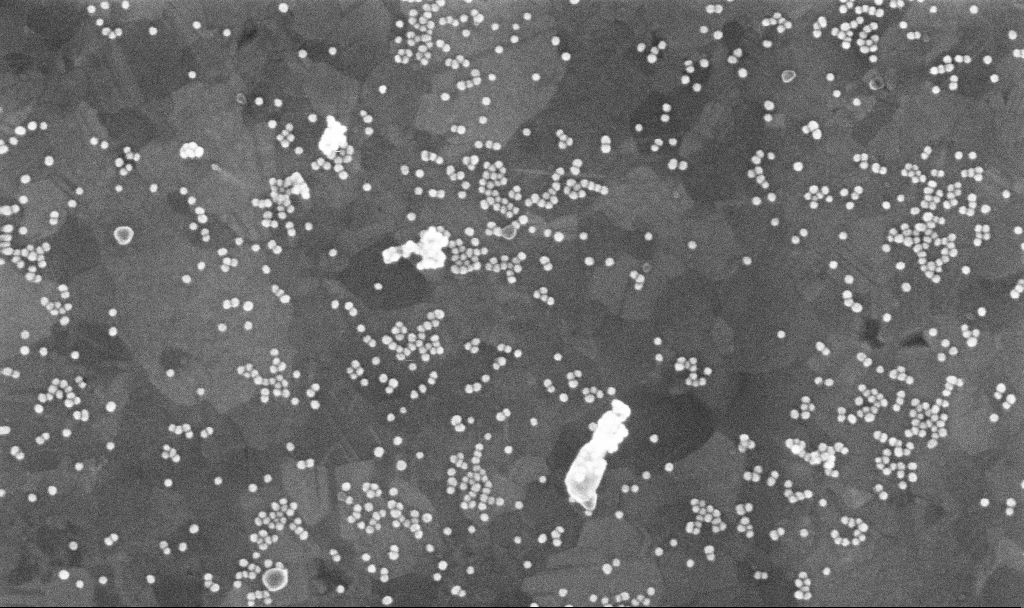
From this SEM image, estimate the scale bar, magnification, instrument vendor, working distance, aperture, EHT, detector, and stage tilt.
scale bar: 200 nm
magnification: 156.42 K X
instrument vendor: Zeiss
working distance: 3.7 mm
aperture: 30 µm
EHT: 10 kV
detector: InLens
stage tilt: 0°